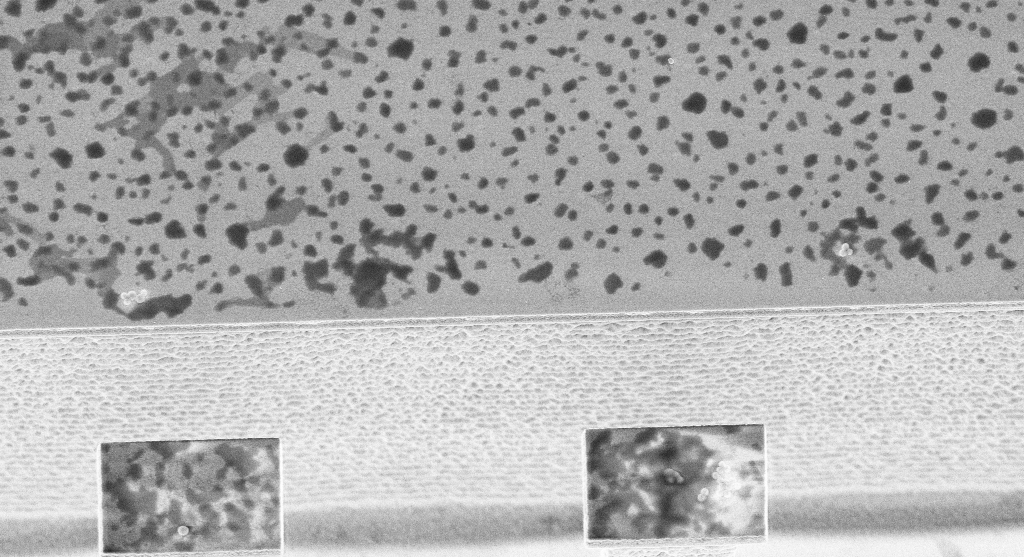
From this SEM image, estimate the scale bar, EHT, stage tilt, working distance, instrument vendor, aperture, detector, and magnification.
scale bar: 2000 nm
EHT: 3 kV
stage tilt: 20°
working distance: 5.6 mm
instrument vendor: Zeiss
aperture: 30 µm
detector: InLens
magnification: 14.76 K X